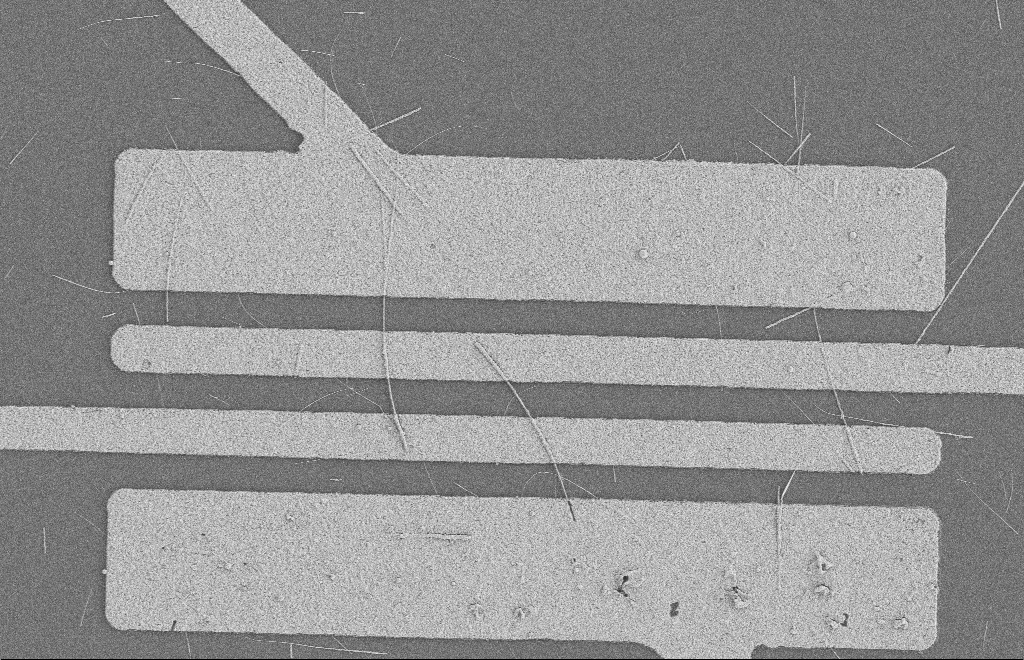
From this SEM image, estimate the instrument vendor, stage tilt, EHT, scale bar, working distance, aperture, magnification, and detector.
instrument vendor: Zeiss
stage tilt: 0°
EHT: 2 kV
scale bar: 2000 nm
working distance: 8 mm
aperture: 20 µm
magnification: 5.01 K X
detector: SE2